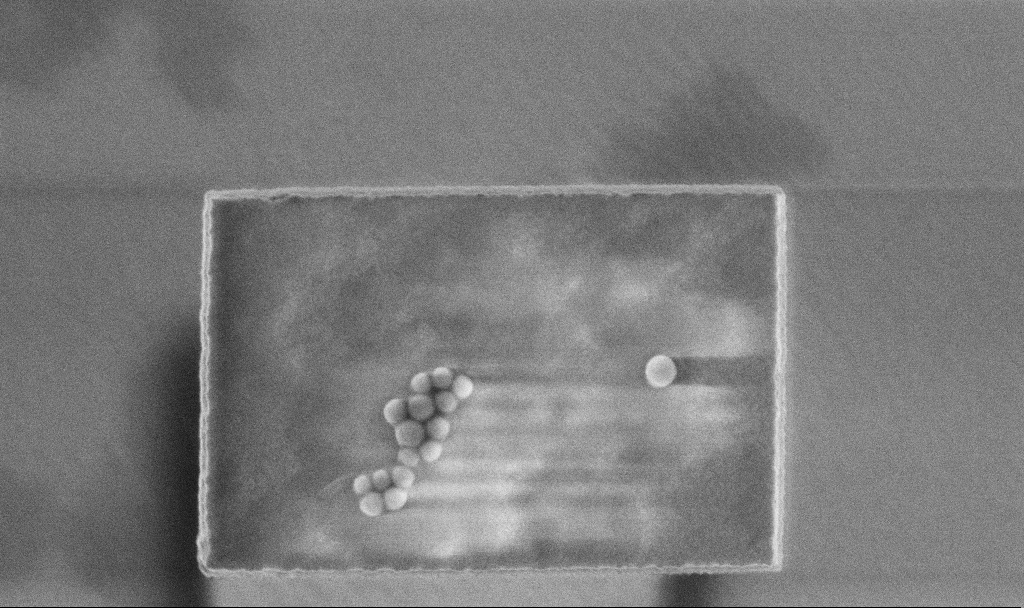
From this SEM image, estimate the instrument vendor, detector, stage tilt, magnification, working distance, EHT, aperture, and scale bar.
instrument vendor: Zeiss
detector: InLens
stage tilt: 0°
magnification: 46.79 K X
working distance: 3.4 mm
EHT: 3 kV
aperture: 30 µm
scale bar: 1000 nm